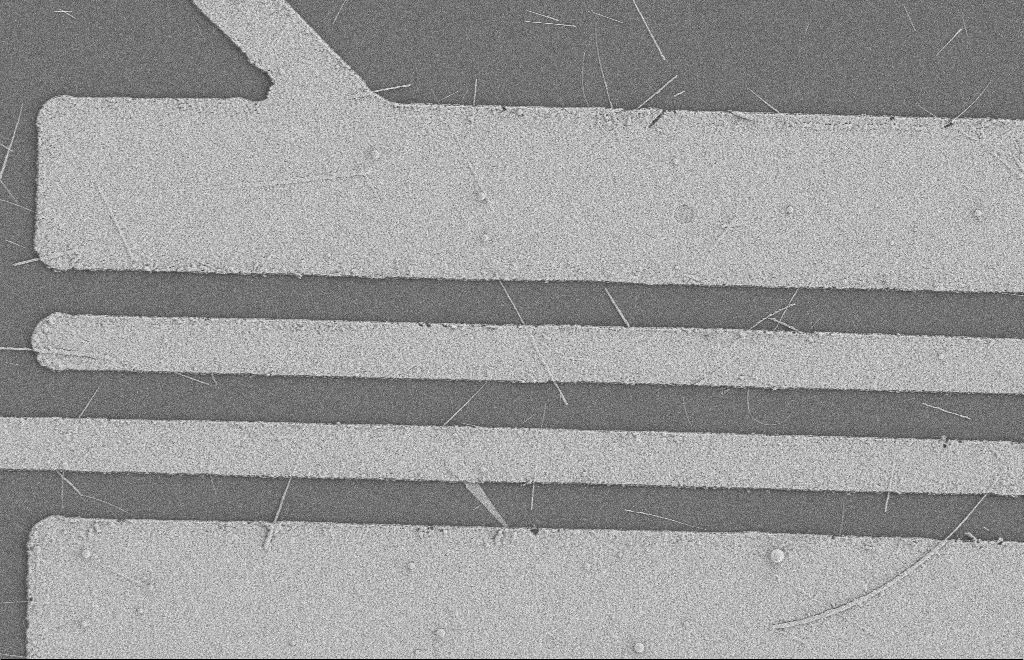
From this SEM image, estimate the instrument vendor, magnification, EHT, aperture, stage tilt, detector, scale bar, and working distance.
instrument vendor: Zeiss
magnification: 6.21 K X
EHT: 2 kV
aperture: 20 µm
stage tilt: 0°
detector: SE2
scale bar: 2000 nm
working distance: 8 mm